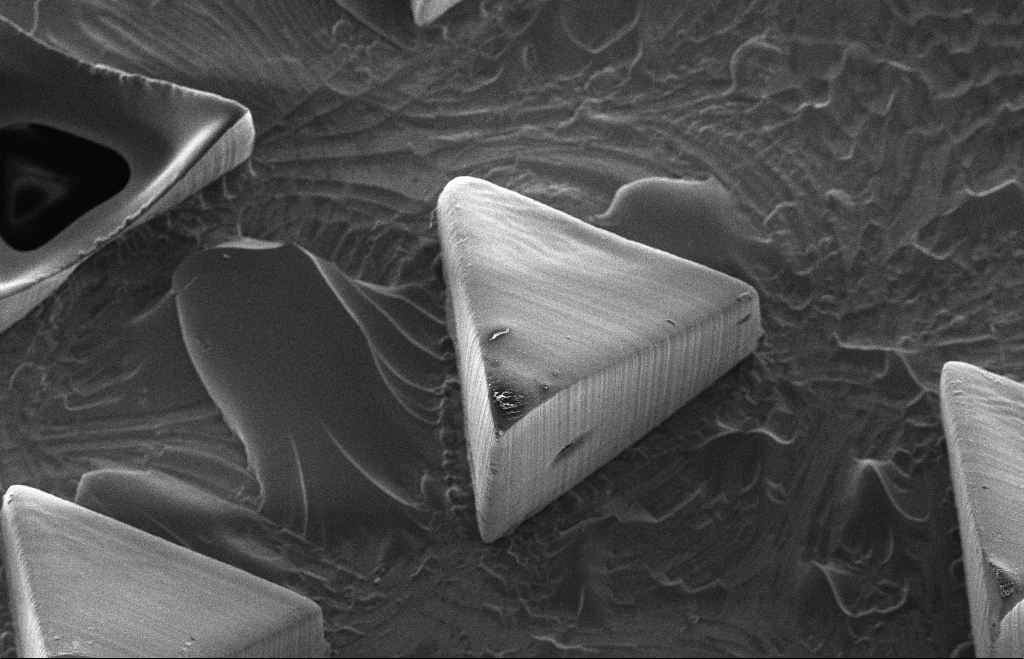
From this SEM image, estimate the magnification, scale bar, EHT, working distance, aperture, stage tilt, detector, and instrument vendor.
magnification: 0.419 K X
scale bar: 100000 nm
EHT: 5 kV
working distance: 14 mm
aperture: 30 µm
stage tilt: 17.3°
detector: SE2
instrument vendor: Zeiss